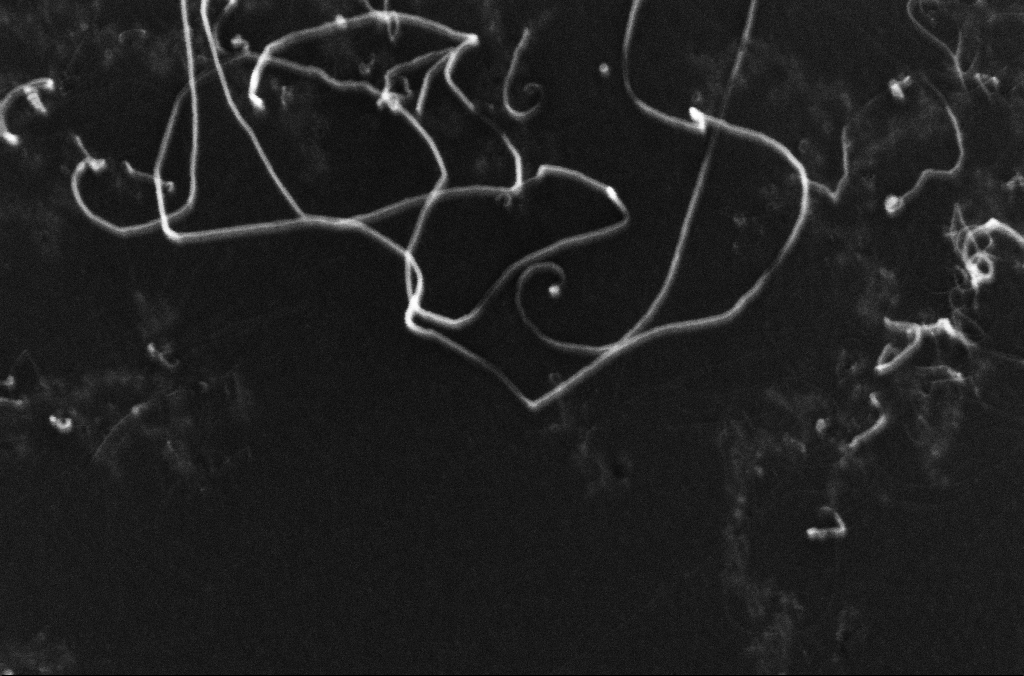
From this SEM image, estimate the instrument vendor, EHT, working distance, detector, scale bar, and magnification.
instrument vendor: Zeiss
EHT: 10 kV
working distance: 3.2 mm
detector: InLens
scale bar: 200 nm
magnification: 200.42 K X